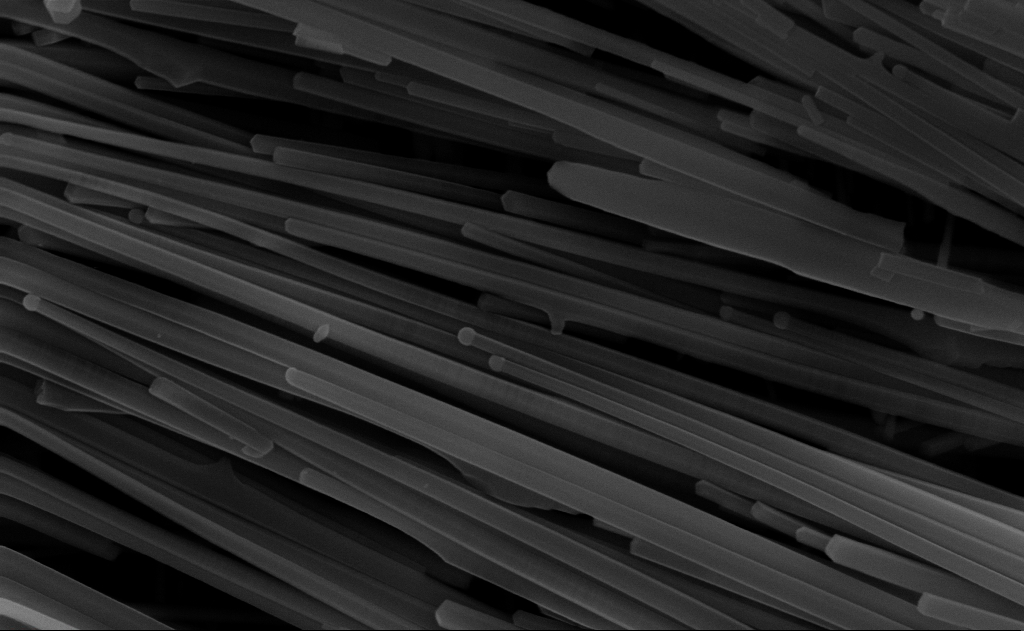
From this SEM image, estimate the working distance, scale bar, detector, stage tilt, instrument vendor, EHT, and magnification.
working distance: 9 mm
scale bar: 200 nm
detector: InLens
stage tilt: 0°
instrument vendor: Zeiss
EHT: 10 kV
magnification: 80 K X